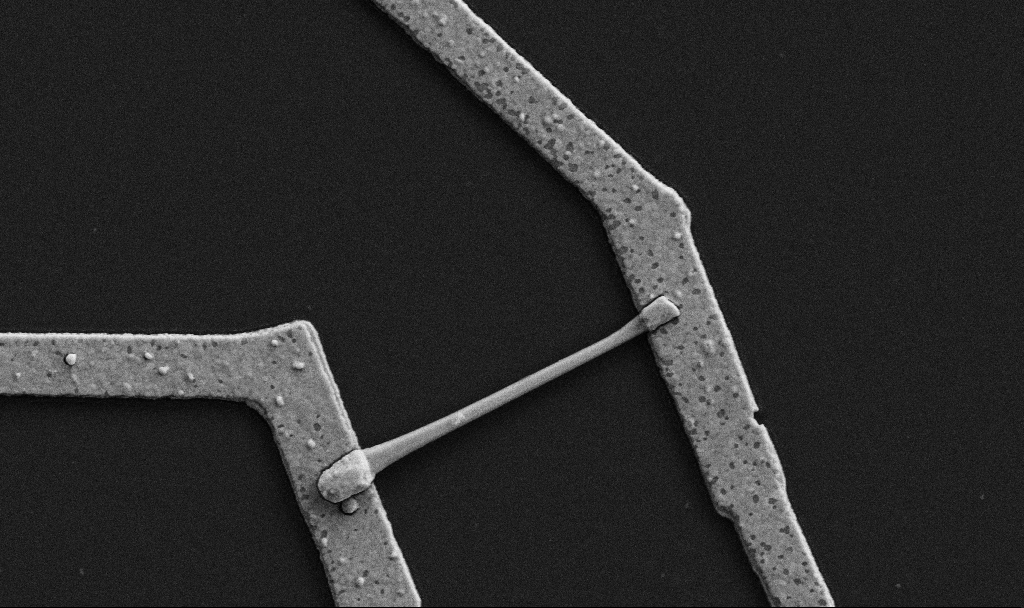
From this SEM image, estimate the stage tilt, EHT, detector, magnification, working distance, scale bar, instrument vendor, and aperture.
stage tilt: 0°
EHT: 5 kV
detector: SE2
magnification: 30 K X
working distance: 8.7 mm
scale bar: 1000 nm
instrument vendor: Zeiss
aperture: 30 µm